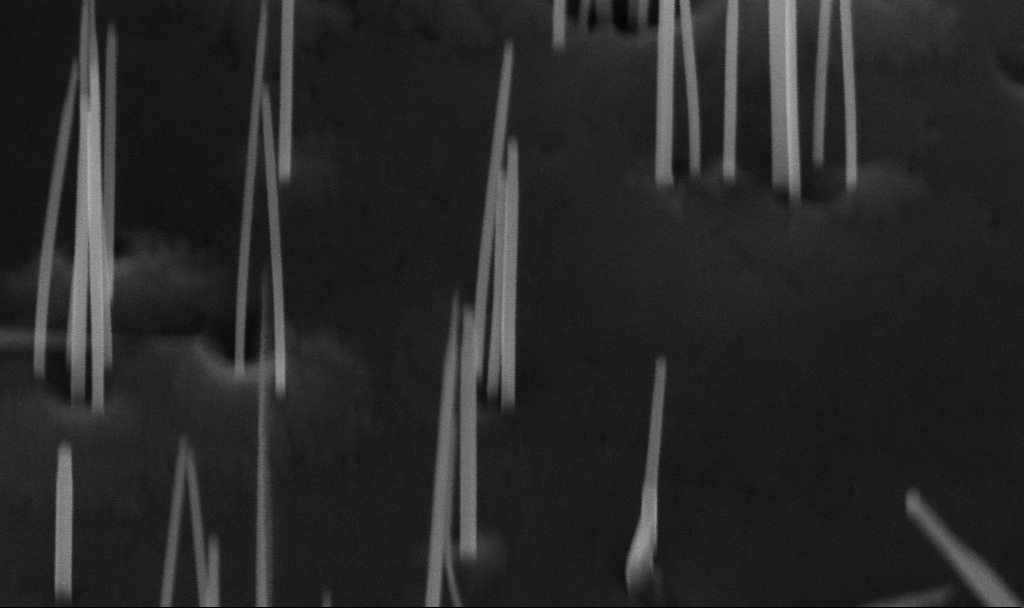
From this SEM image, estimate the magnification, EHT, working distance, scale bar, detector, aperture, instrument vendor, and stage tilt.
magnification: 171.08 K X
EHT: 10 kV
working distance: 5.6 mm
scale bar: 200 nm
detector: InLens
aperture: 30 µm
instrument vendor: Zeiss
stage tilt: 45°